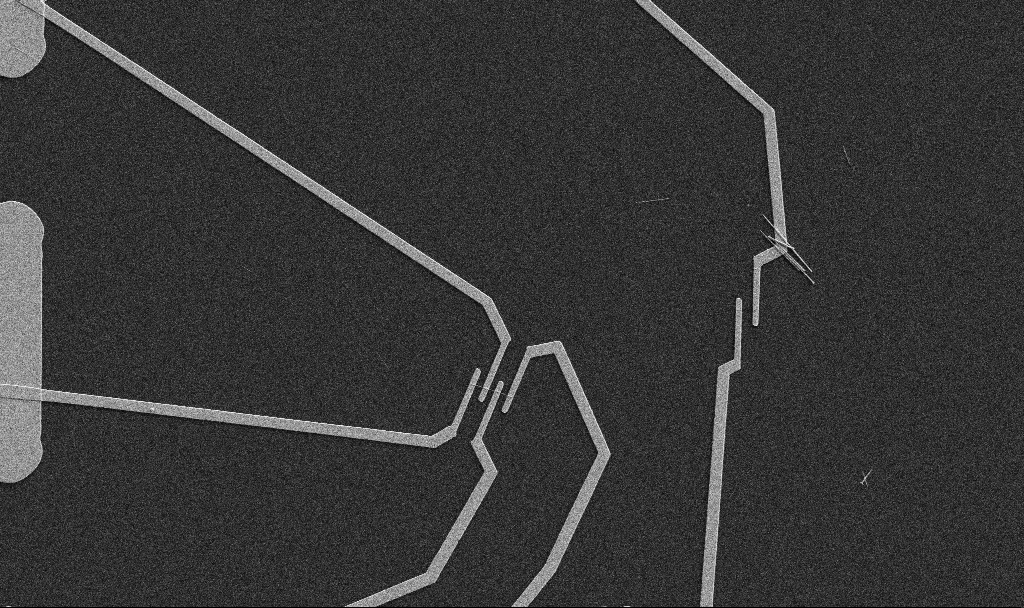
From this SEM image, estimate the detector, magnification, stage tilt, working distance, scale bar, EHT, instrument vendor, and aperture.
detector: SE2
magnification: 5 K X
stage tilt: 0°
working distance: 10.7 mm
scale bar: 10000 nm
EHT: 5 kV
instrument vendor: Zeiss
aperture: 30 µm